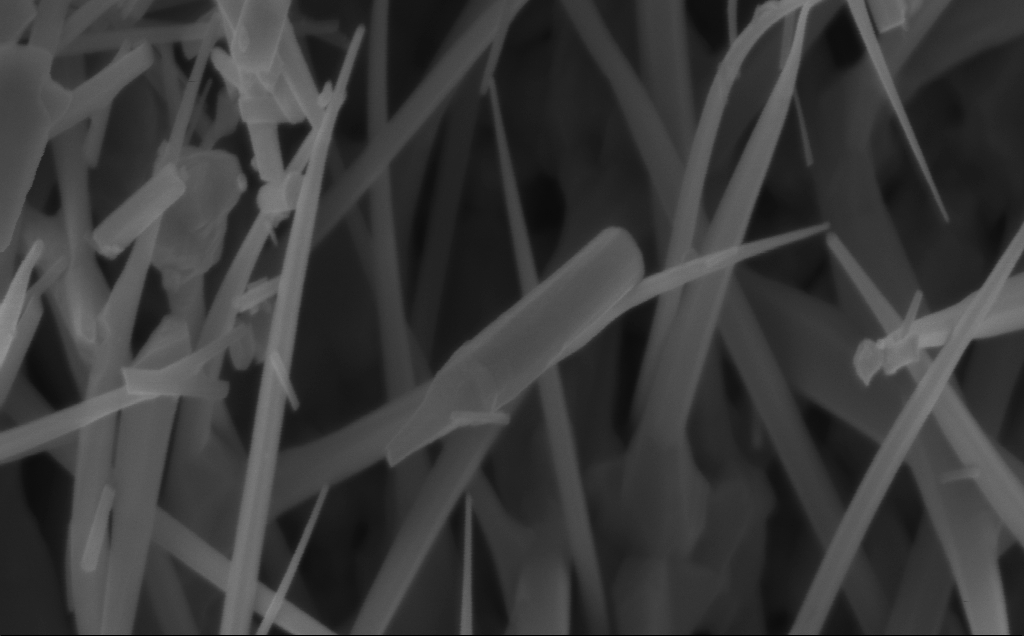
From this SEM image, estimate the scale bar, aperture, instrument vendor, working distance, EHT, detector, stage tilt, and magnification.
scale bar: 200 nm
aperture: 30 µm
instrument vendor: Zeiss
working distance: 5 mm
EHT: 5 kV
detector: InLens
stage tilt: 0°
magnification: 126.17 K X